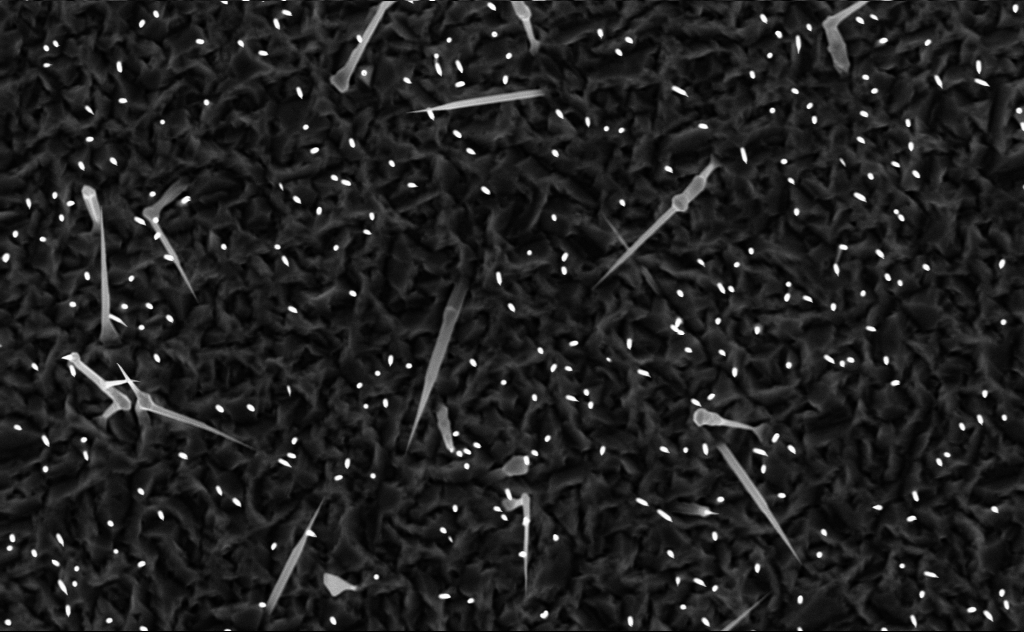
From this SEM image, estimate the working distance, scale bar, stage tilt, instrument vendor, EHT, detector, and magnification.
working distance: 6 mm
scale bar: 2000 nm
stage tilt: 0°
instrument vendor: Zeiss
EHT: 10 kV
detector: InLens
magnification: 10 K X